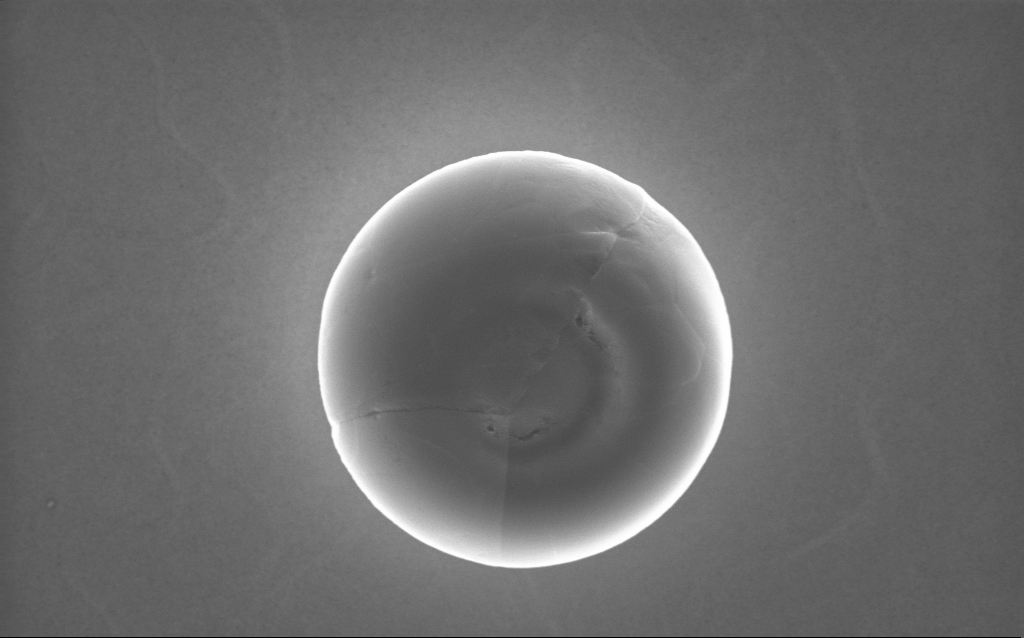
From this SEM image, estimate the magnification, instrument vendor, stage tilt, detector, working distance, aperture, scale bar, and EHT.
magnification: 40.9 K X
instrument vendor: Zeiss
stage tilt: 0°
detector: InLens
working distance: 2 mm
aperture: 30 µm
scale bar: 1000 nm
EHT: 10 kV